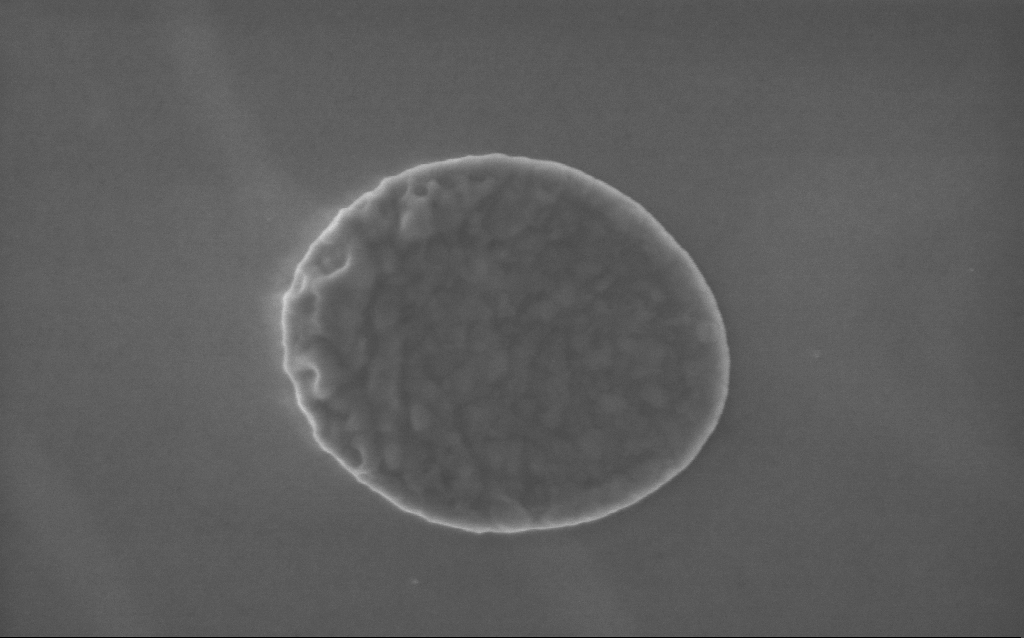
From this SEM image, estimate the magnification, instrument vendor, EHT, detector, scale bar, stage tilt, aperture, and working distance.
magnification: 91 K X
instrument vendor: Zeiss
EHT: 5 kV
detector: InLens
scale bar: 200 nm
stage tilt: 0°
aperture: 30 µm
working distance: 4 mm